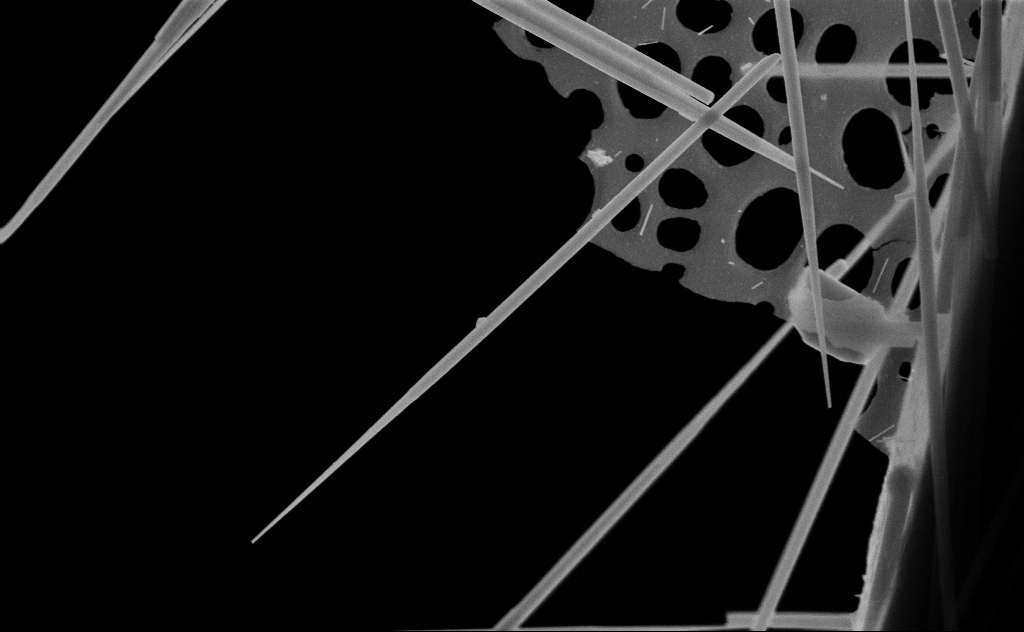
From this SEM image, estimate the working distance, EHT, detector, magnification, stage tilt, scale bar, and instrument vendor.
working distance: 10 mm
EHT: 20 kV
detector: SE2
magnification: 37.51 K X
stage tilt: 0°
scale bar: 1000 nm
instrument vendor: Zeiss